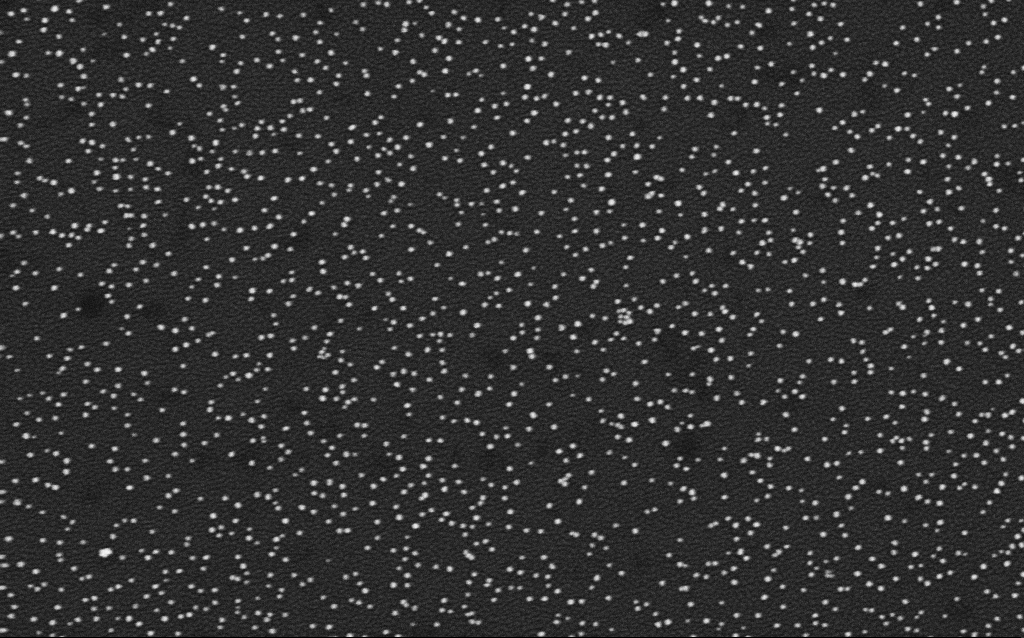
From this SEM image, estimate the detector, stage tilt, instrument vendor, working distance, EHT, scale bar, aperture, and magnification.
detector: InLens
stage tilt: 0°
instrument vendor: Zeiss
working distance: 2.1 mm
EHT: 4 kV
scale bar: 200 nm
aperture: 30 µm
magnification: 100 K X